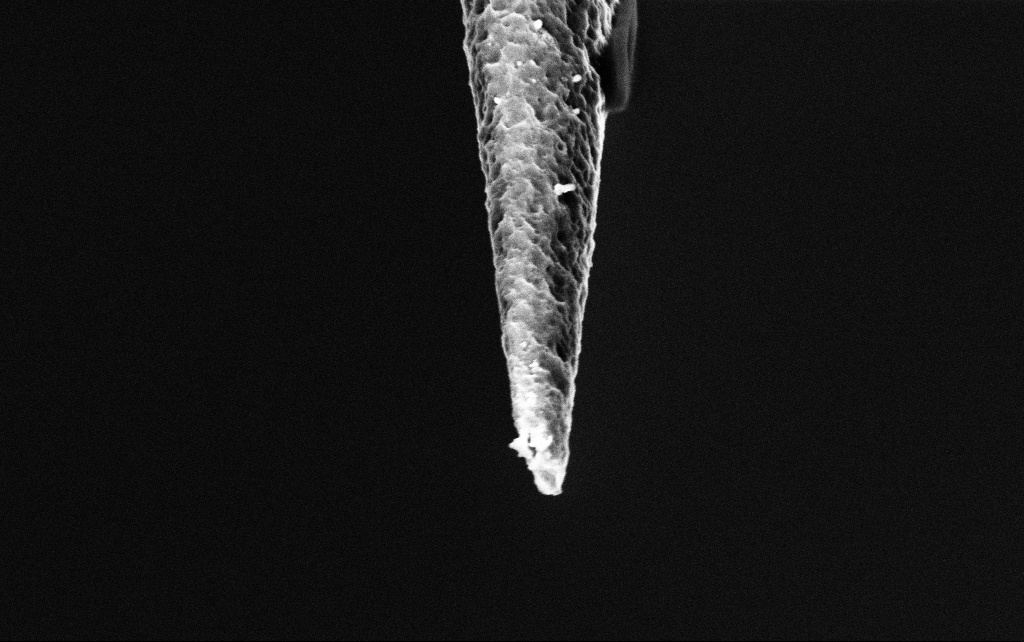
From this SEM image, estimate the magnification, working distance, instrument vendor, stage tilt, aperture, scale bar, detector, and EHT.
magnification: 50 K X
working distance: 7.7 mm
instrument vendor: Zeiss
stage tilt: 45°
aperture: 30 µm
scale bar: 1000 nm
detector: InLens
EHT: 3 kV